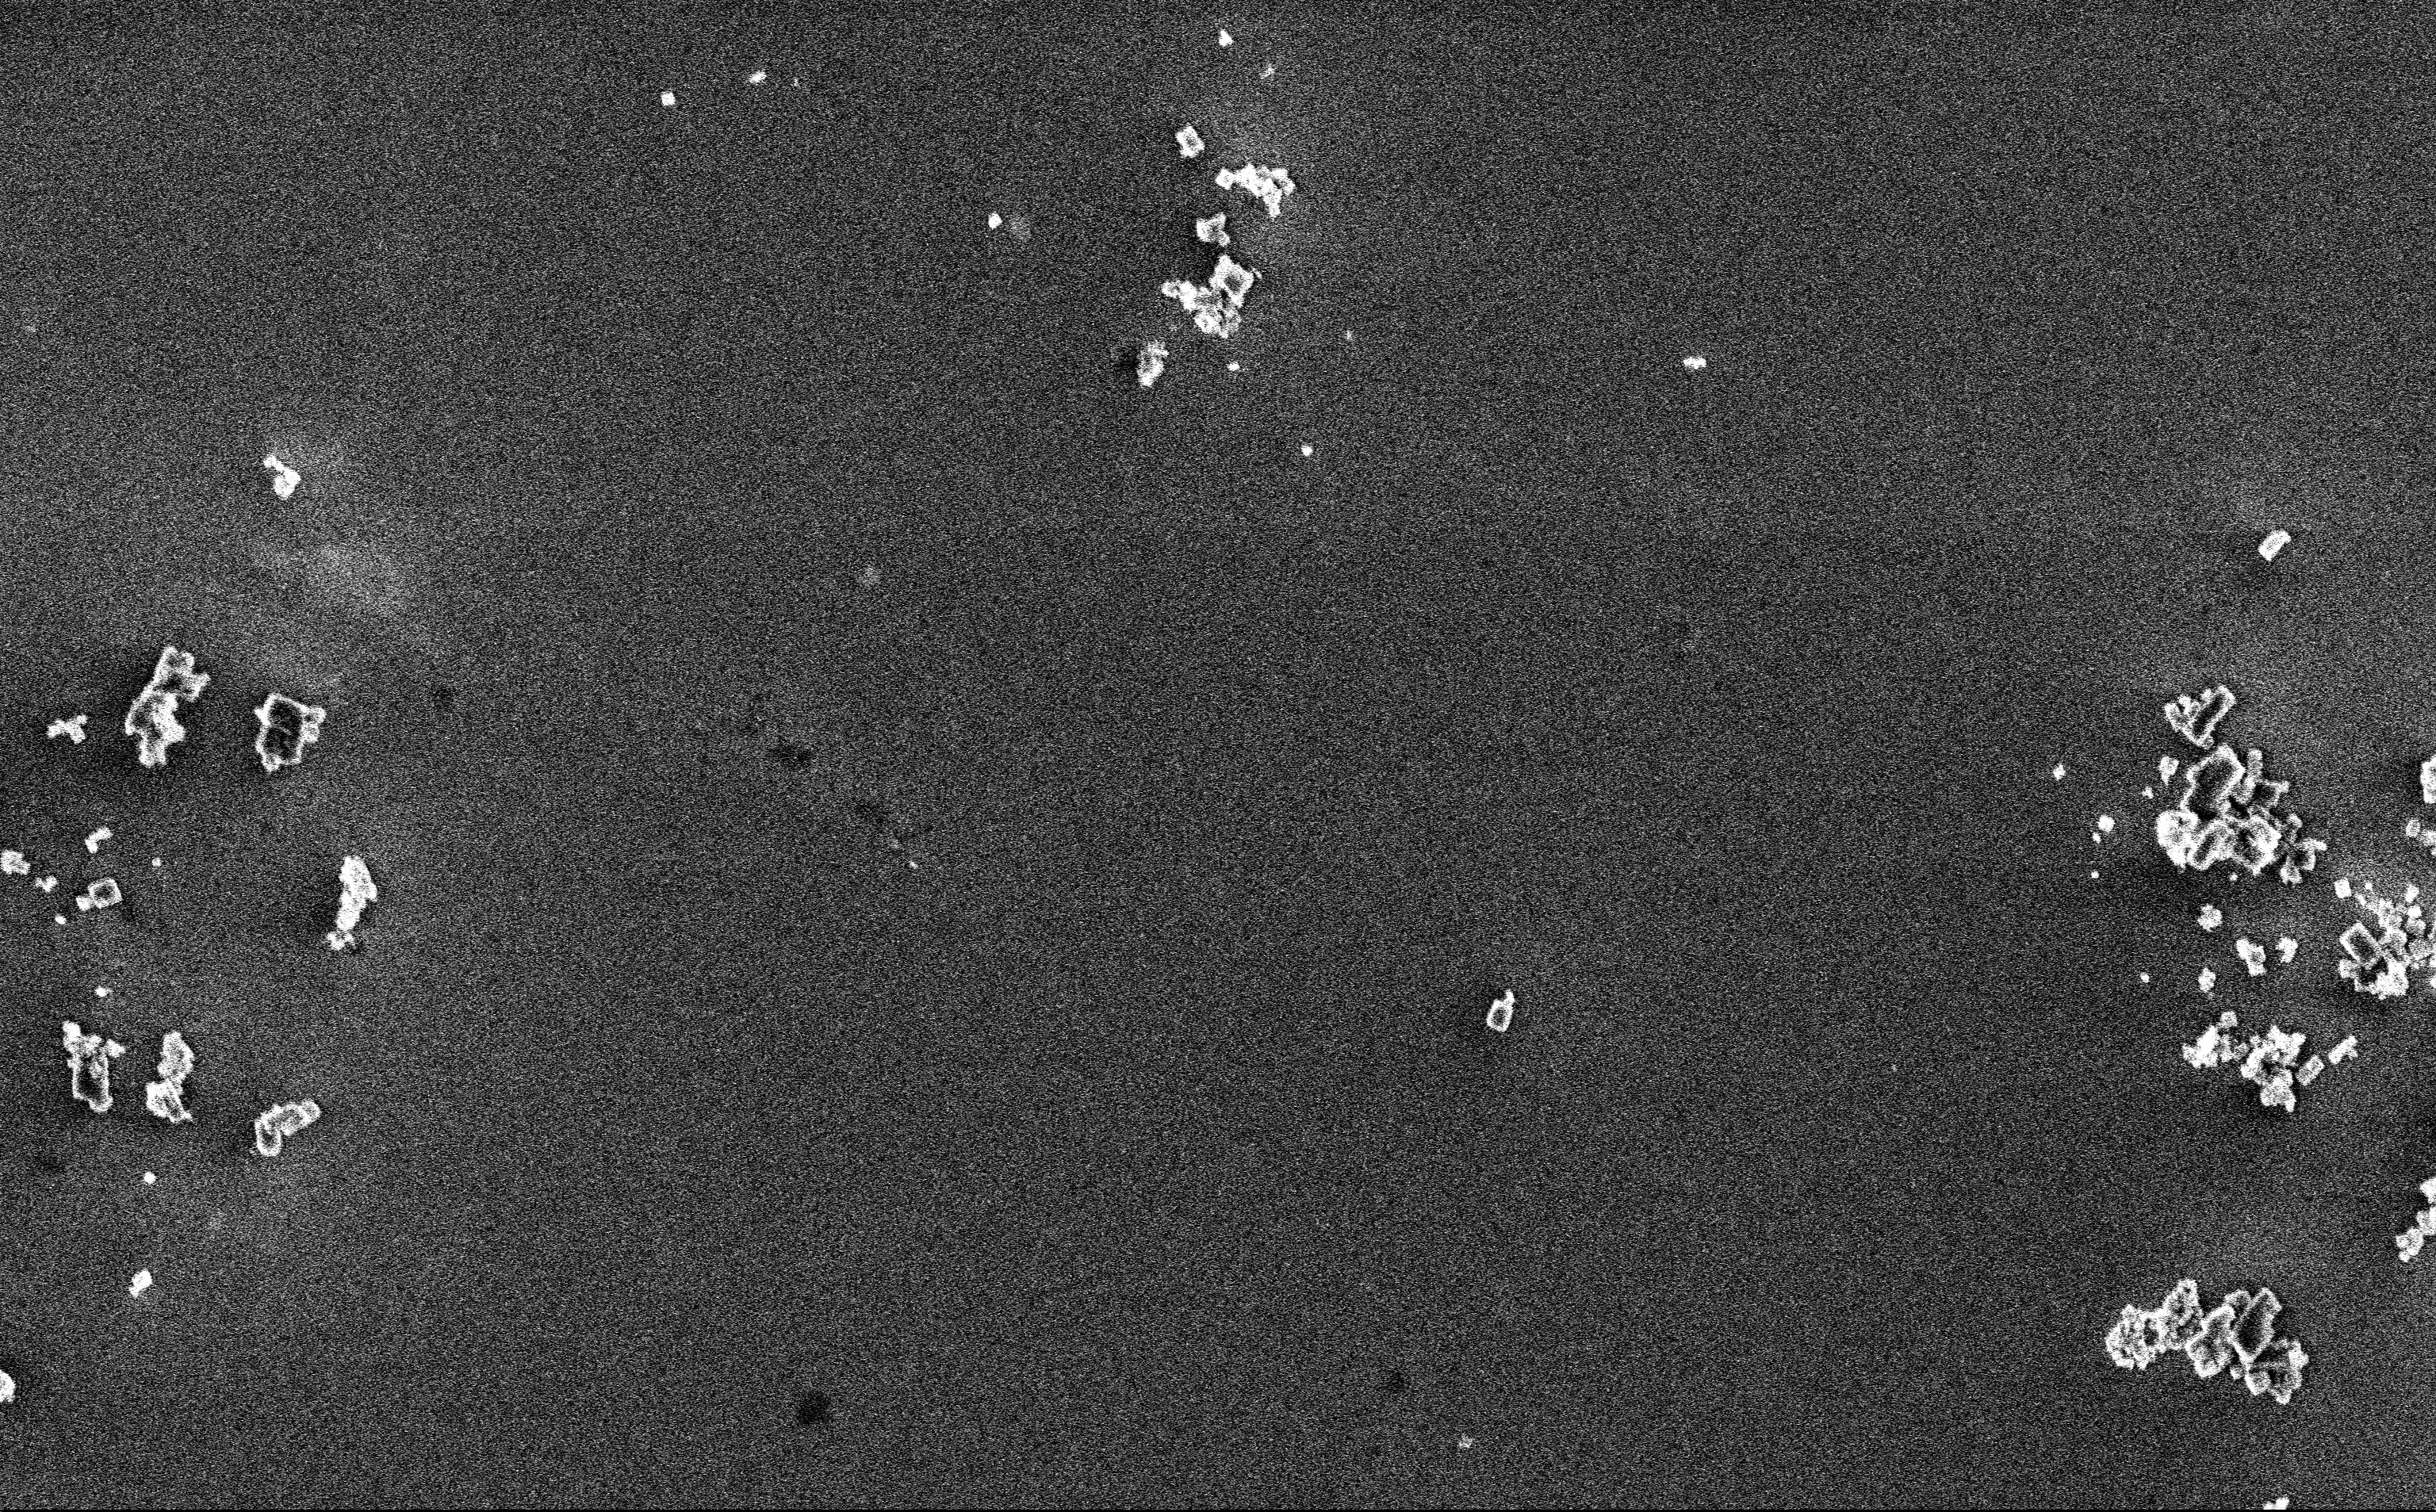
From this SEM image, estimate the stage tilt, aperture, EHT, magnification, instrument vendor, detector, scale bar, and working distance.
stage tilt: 0°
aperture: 30 µm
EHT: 3 kV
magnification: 12.85 K X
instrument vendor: Zeiss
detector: InLens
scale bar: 2000 nm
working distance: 3 mm